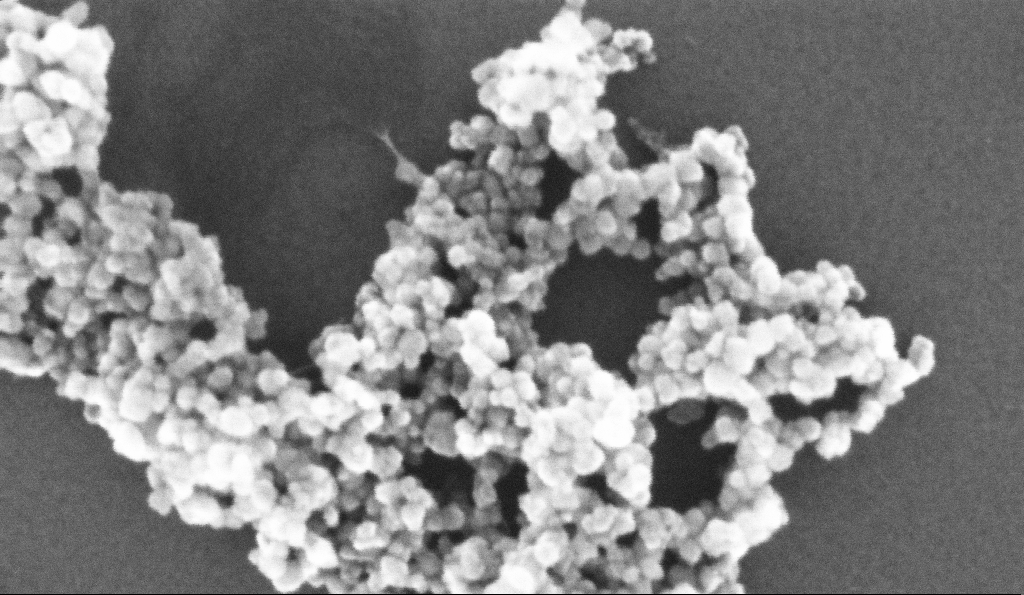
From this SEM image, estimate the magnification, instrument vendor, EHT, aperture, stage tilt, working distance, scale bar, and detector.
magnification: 450.71 K X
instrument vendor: Zeiss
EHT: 10 kV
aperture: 30 µm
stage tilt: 0°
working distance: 5.2 mm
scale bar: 100 nm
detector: InLens